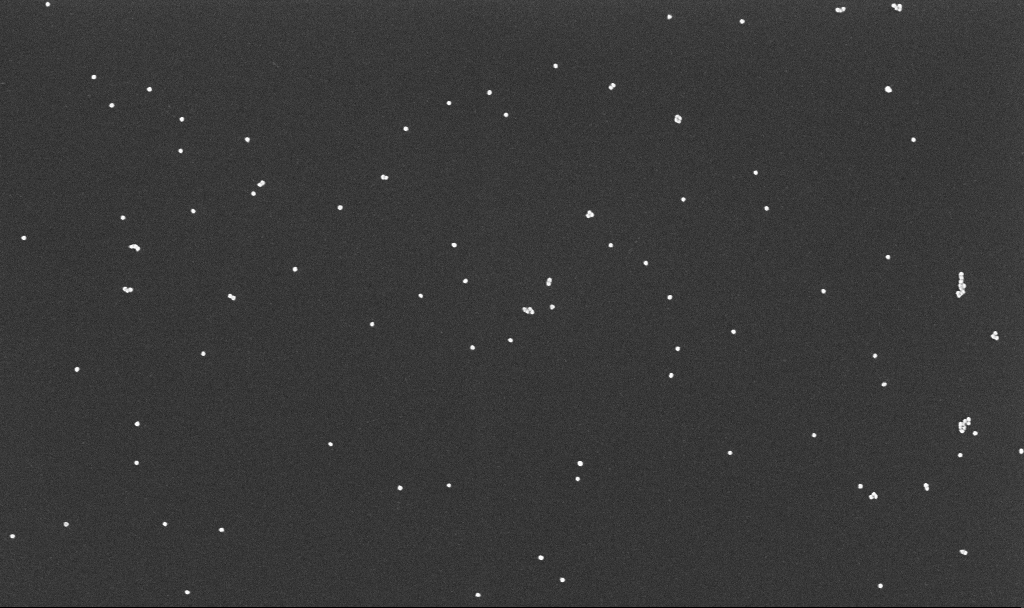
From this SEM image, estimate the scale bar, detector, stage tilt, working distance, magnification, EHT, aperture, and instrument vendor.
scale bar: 1000 nm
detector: InLens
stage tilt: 0°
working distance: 3.4 mm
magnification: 70 K X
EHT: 10 kV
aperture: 30 µm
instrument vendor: Zeiss